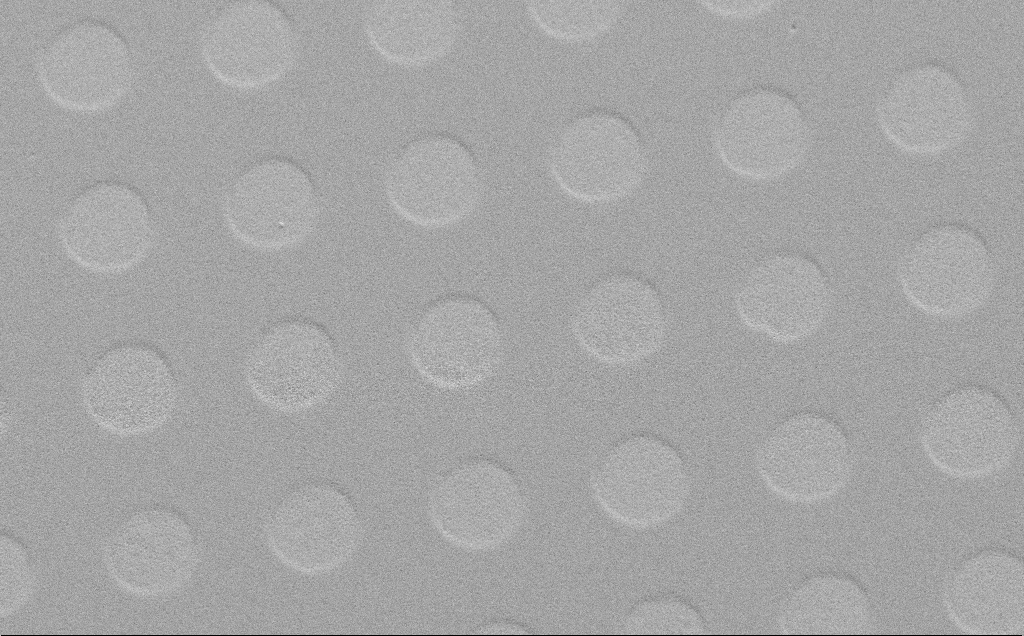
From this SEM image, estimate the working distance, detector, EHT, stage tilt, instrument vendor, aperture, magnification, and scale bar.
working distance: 6 mm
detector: SE2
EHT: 10 kV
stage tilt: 40.4°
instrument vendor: Zeiss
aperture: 30 µm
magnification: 2.03 K X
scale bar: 10000 nm